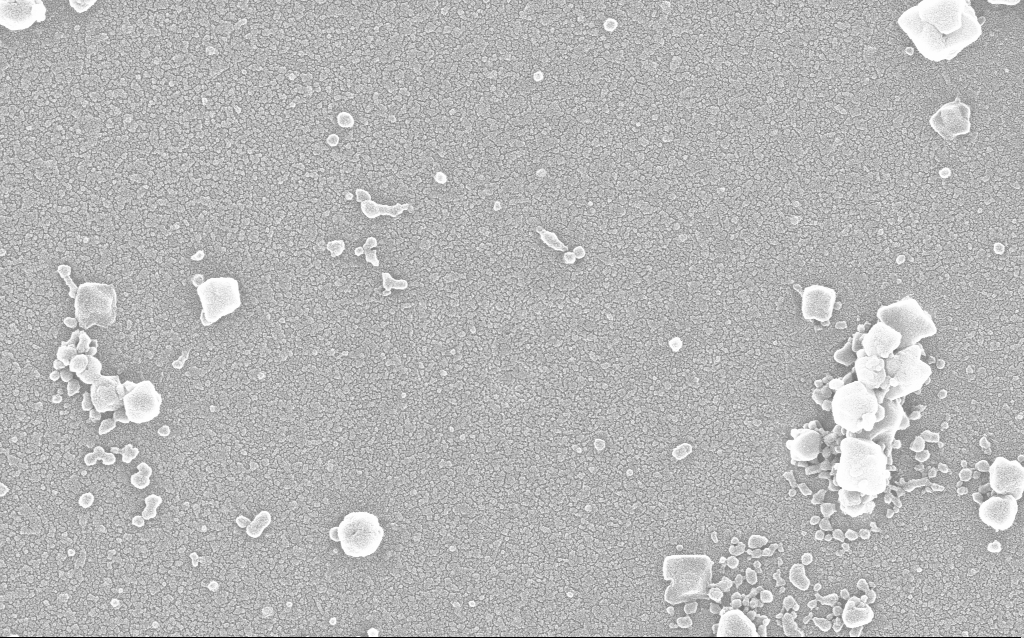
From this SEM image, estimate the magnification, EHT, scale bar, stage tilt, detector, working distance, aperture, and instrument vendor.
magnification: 100 K X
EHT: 20 kV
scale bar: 200 nm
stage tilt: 0°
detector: InLens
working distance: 1.9 mm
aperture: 30 µm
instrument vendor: Zeiss